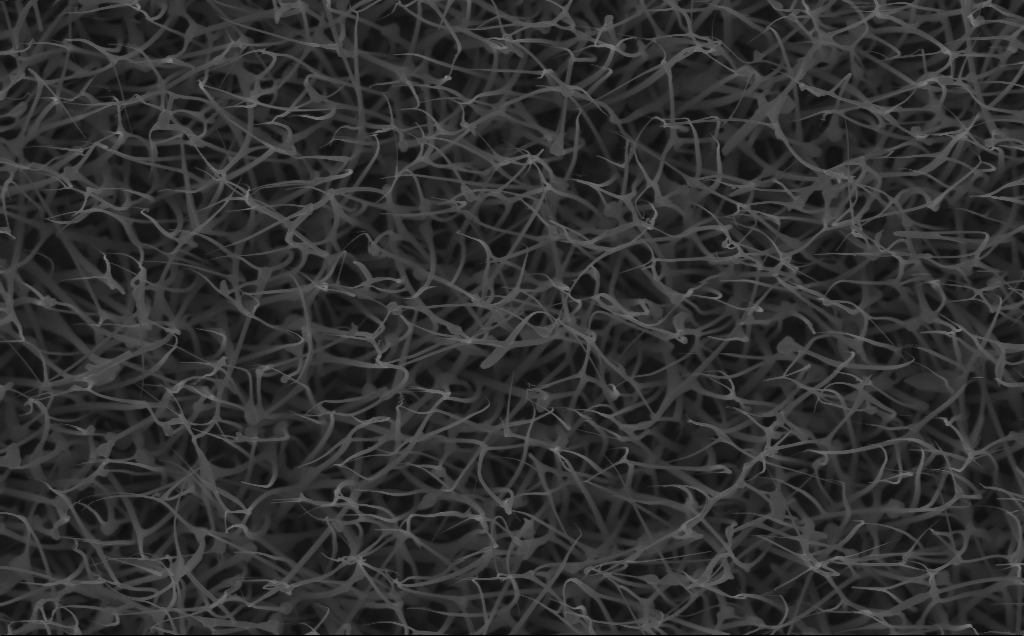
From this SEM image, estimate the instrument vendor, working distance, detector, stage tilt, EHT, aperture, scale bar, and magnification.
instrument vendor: Zeiss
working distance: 6 mm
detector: InLens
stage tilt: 0°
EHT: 10 kV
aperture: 30 µm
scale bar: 2000 nm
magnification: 20 K X